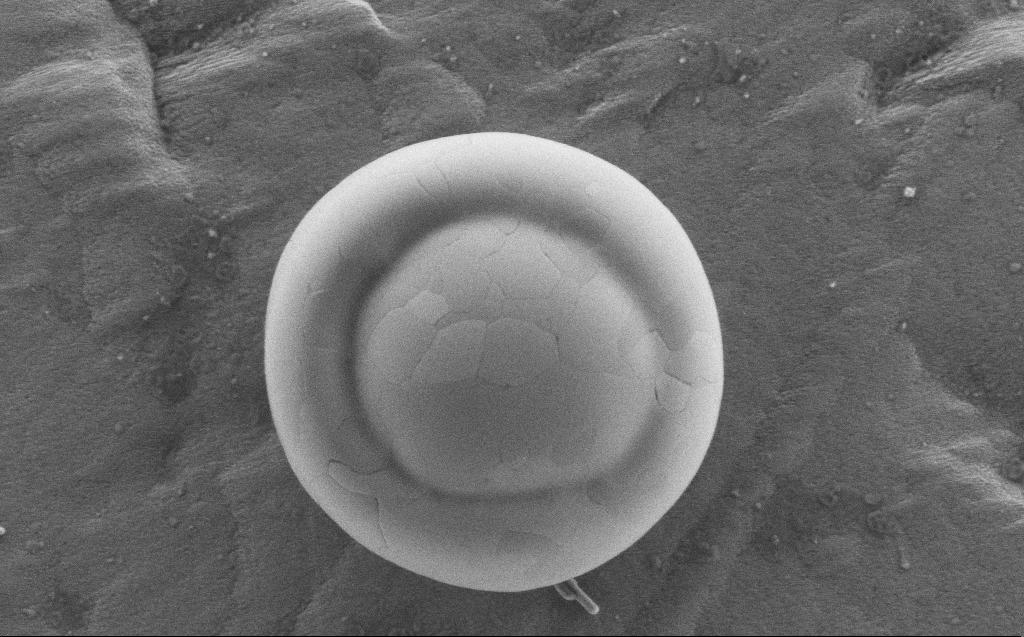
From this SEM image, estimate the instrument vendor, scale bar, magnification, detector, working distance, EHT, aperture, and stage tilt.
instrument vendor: Zeiss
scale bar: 1000 nm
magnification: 40.12 K X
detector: SE2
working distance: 2 mm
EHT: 2 kV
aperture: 30 µm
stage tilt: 0°